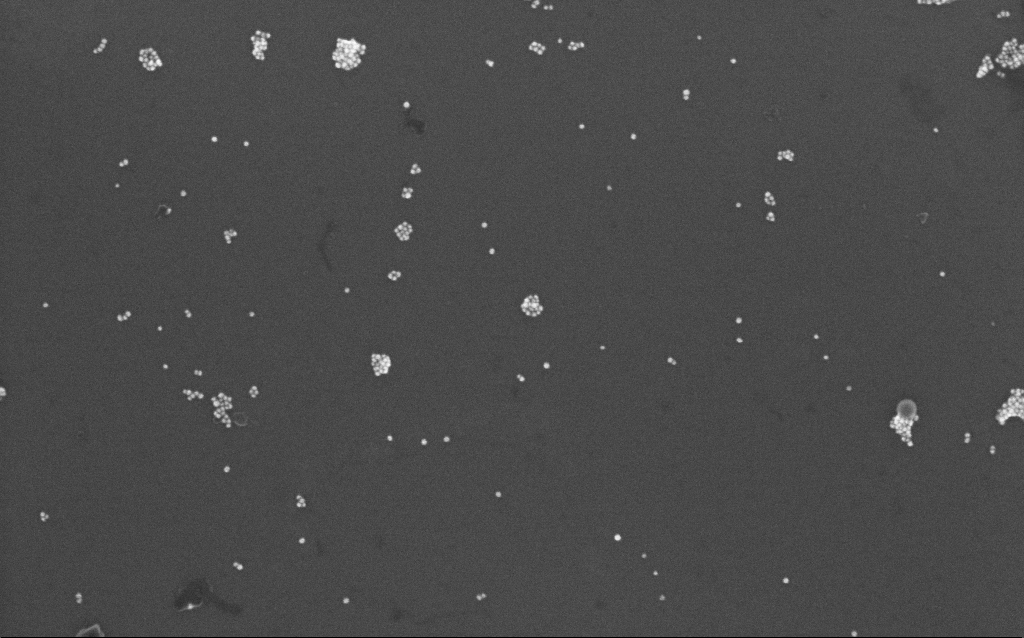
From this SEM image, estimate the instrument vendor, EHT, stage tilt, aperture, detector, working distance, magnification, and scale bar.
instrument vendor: Zeiss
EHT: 10 kV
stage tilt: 0°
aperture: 30 µm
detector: InLens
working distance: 7 mm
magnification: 100 K X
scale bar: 200 nm